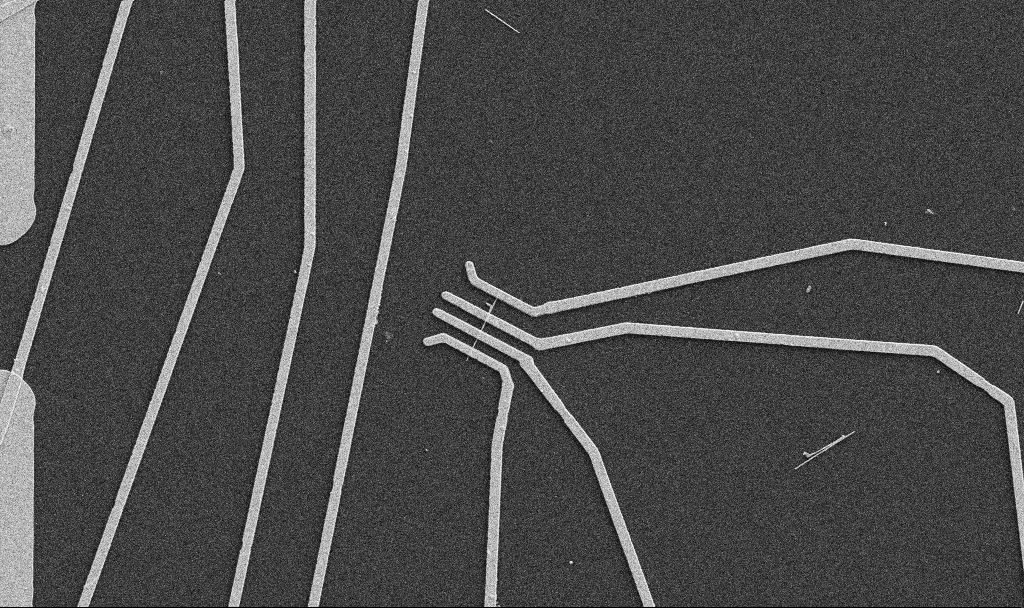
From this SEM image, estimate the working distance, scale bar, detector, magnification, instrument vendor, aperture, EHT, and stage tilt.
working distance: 10.7 mm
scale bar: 10000 nm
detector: SE2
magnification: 5 K X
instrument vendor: Zeiss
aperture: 30 µm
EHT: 5 kV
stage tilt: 0°